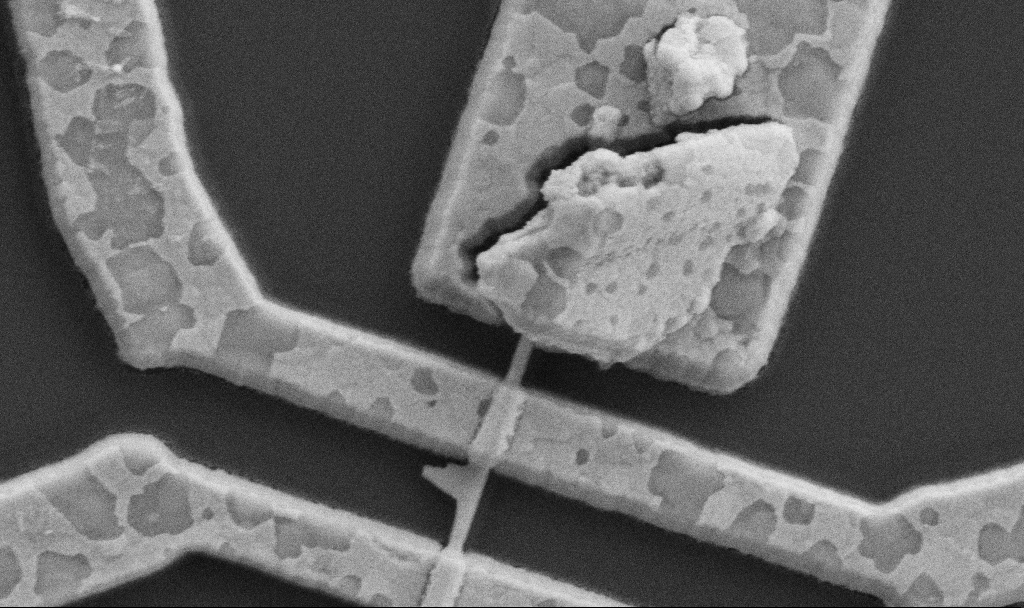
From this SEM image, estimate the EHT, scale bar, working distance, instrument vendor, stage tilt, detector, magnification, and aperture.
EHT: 5 kV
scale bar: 1000 nm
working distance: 8.7 mm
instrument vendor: Zeiss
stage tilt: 0°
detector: SE2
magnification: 60 K X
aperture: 30 µm